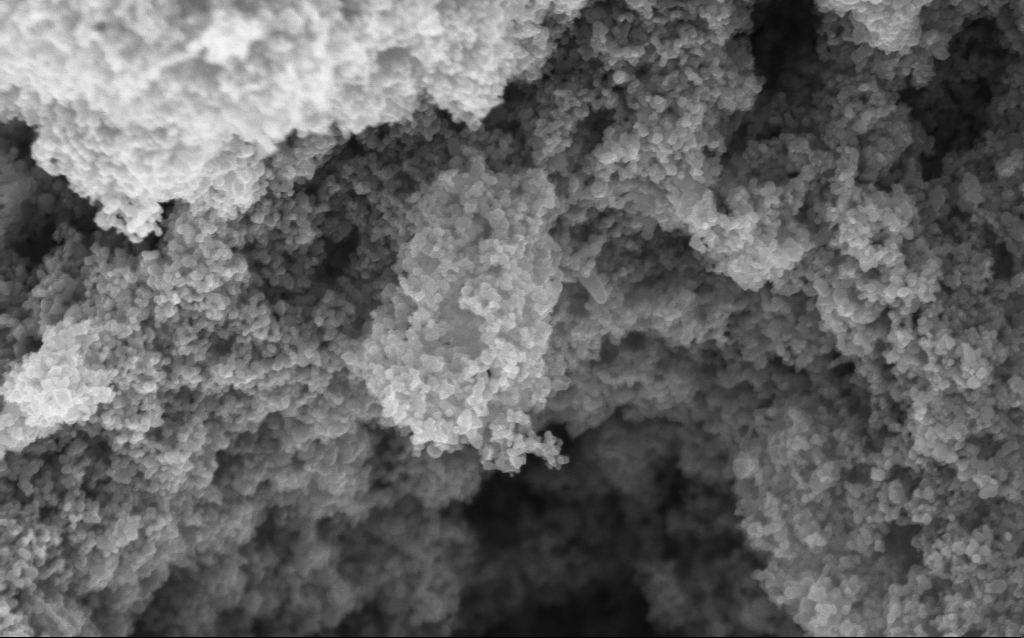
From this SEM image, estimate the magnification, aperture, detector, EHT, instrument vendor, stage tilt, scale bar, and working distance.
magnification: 114.68 K X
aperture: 30 µm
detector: InLens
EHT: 5 kV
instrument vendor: Zeiss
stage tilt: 0°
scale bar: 200 nm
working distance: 4.4 mm